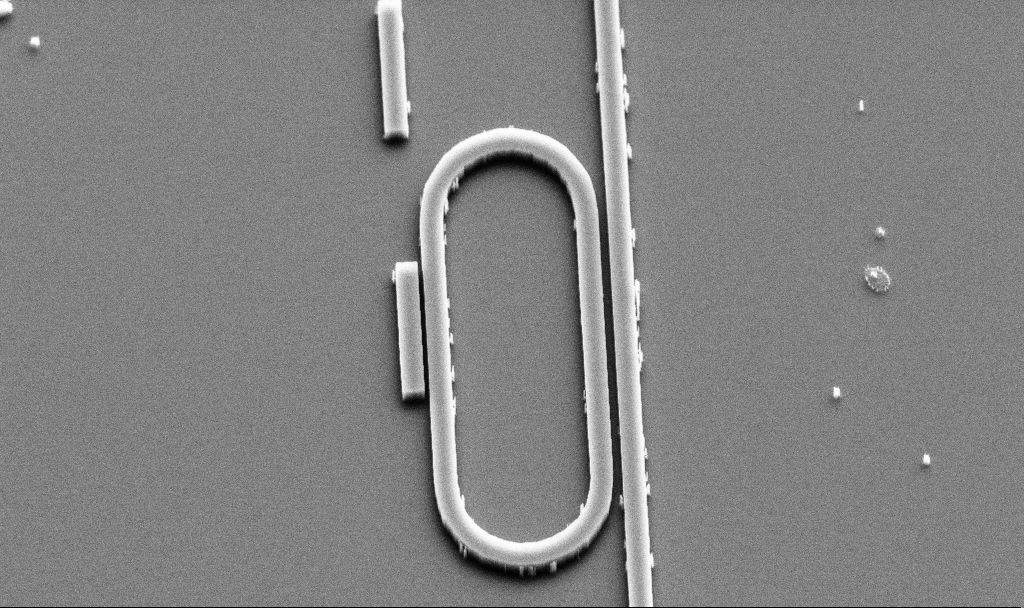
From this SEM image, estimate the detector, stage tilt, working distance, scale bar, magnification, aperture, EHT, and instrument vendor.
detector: SE2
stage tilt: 45°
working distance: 10 mm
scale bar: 1000 nm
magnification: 16.38 K X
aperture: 30 µm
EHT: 5 kV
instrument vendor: Zeiss